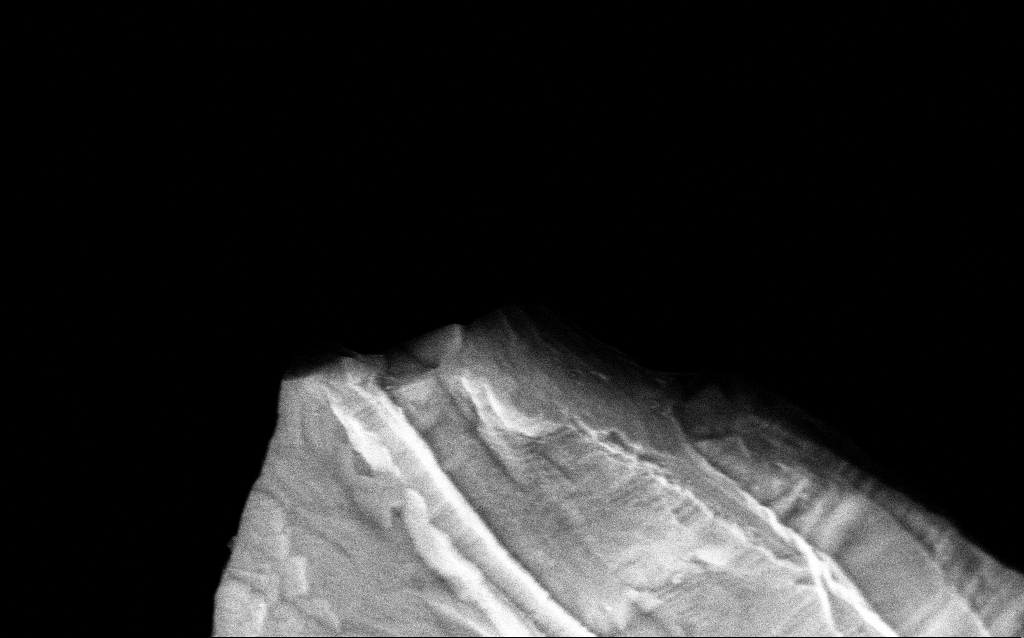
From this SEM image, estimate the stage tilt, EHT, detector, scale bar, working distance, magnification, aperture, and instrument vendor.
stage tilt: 45°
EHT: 10 kV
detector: InLens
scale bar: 200 nm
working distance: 4.3 mm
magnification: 88.13 K X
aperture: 30 µm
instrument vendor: Zeiss